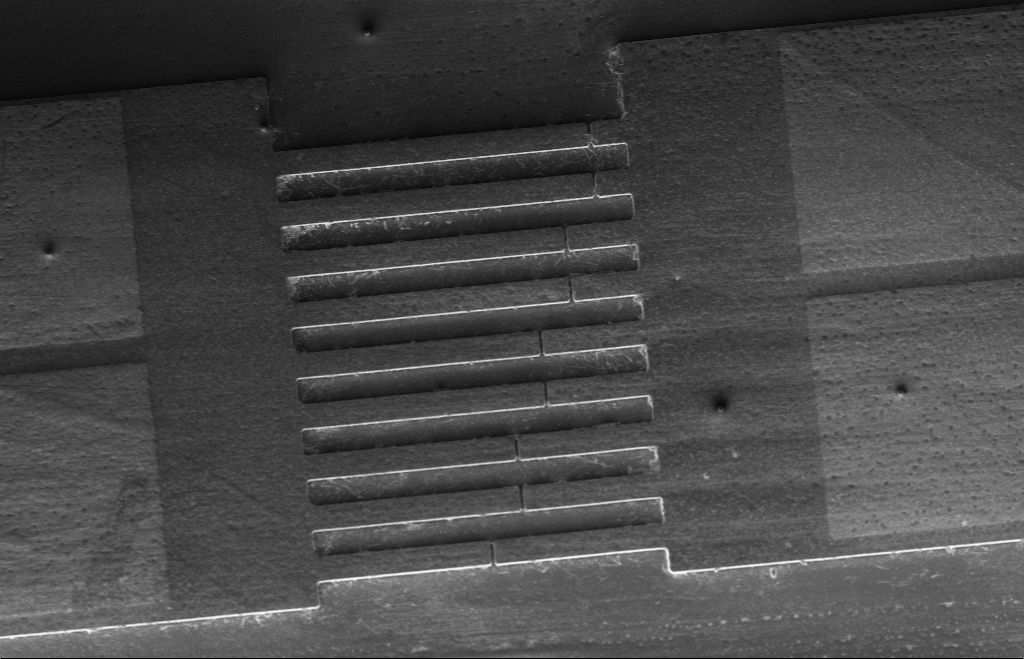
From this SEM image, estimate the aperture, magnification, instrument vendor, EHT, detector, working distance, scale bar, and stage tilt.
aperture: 30 µm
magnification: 0.516 K X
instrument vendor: Zeiss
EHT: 5 kV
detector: InLens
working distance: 9 mm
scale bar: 20000 nm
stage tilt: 23.1°